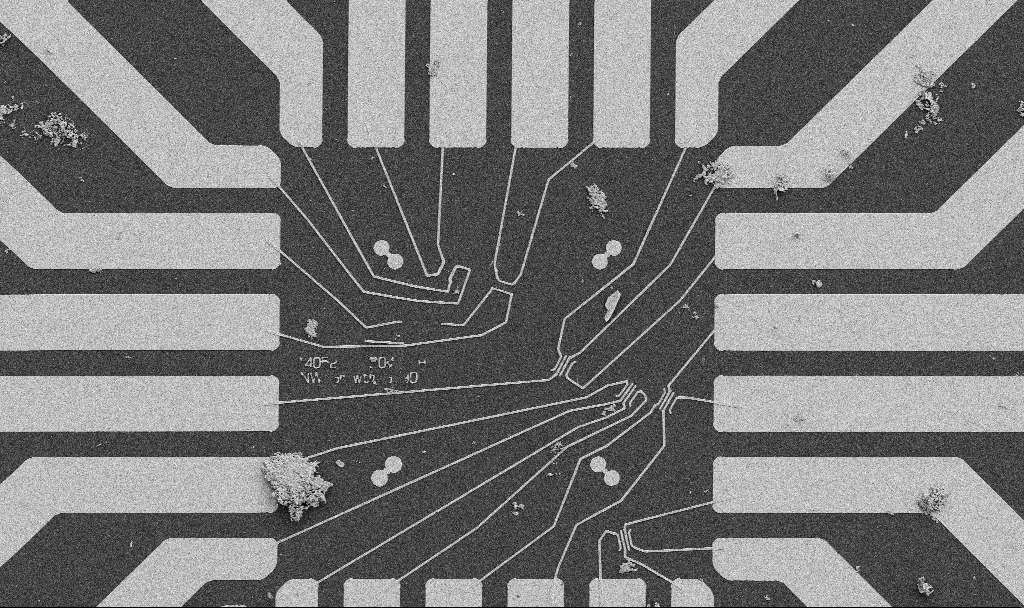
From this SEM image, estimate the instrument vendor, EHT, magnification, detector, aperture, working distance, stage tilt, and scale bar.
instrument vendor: Zeiss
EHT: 5 kV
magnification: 1 K X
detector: SE2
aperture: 30 µm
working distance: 10.7 mm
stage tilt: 0°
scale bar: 20000 nm